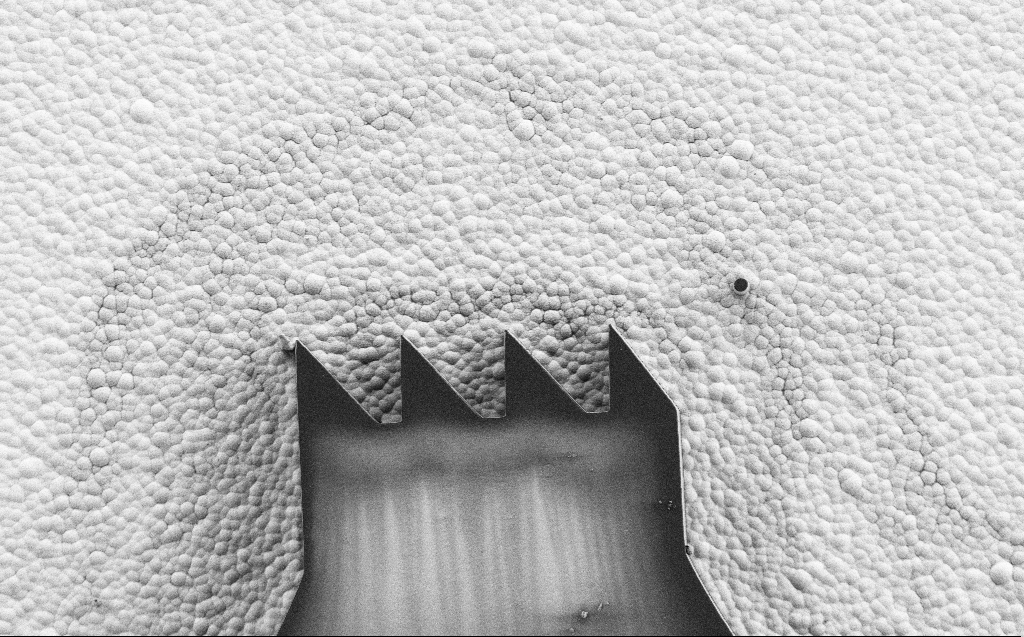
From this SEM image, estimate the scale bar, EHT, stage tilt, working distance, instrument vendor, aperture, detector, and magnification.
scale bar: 10000 nm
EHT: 1 kV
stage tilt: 0°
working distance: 6 mm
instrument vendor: Zeiss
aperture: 30 µm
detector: SE2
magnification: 3.32 K X